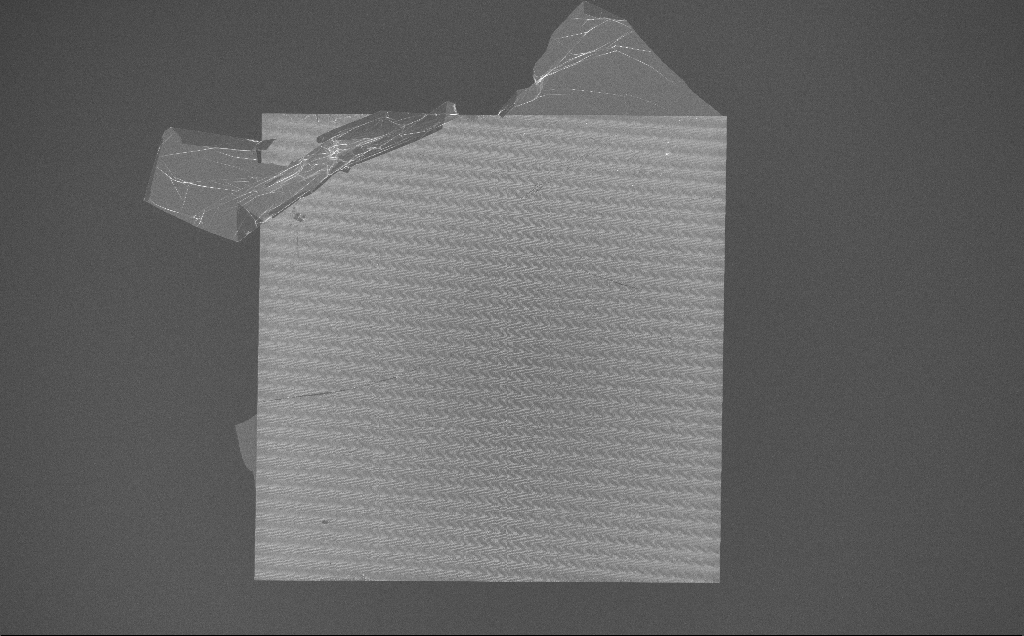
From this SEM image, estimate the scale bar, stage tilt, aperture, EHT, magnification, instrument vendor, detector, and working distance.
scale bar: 100000 nm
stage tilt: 0°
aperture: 30 µm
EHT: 10 kV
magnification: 0.176 K X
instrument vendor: Zeiss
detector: InLens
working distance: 7 mm